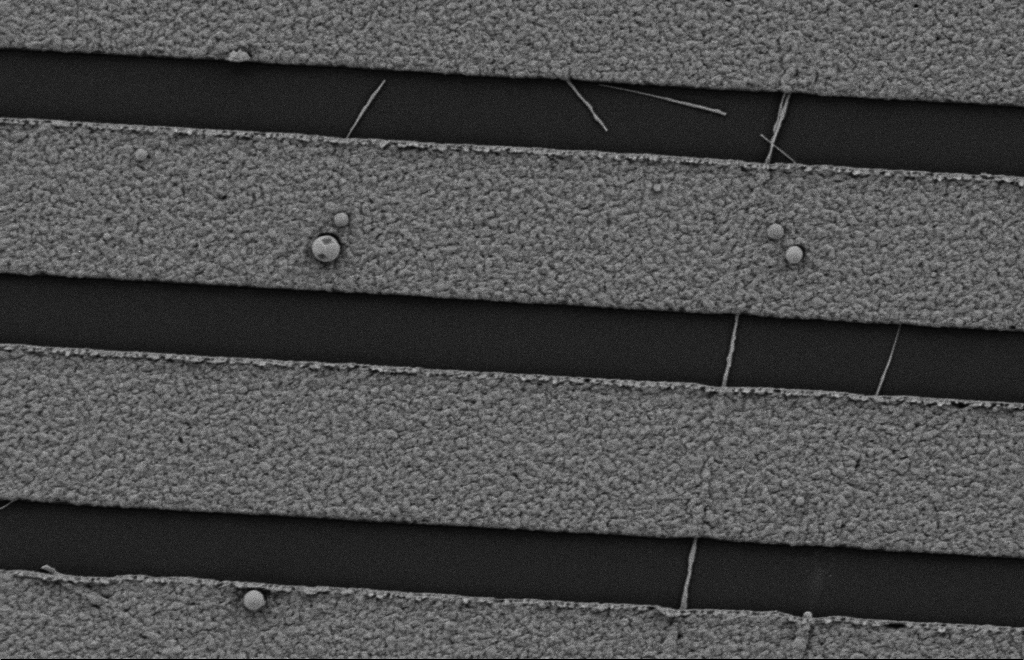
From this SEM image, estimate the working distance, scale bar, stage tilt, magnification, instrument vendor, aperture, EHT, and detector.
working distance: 10 mm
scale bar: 2000 nm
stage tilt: -0.3°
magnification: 20.75 K X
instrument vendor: Zeiss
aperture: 20 µm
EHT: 2 kV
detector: SE2